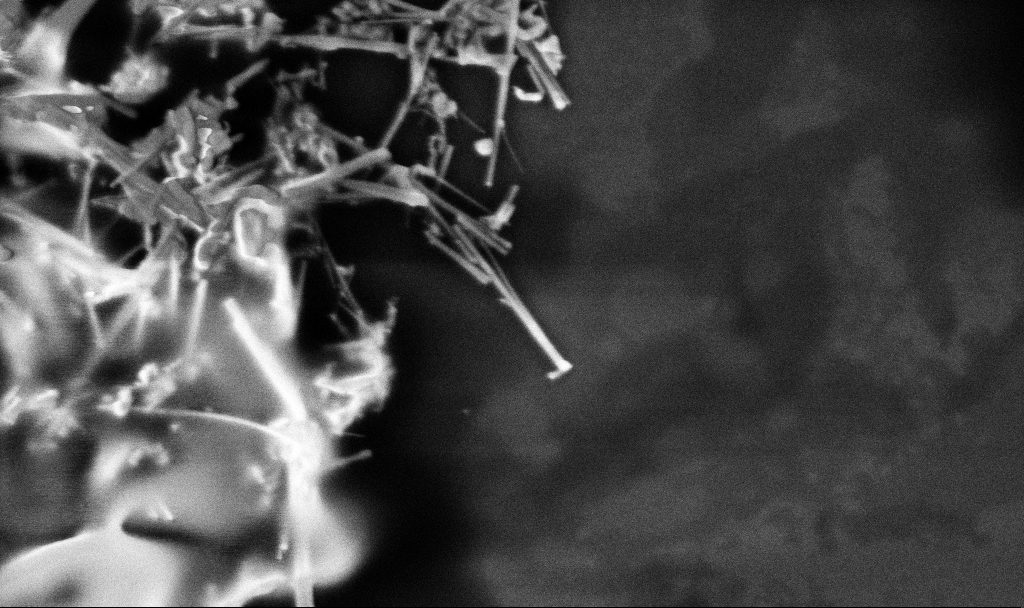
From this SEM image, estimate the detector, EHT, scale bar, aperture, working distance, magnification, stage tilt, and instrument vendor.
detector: InLens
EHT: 3 kV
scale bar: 1000 nm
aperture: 30 µm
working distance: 3.3 mm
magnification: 58.17 K X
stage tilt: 0°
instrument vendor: Zeiss